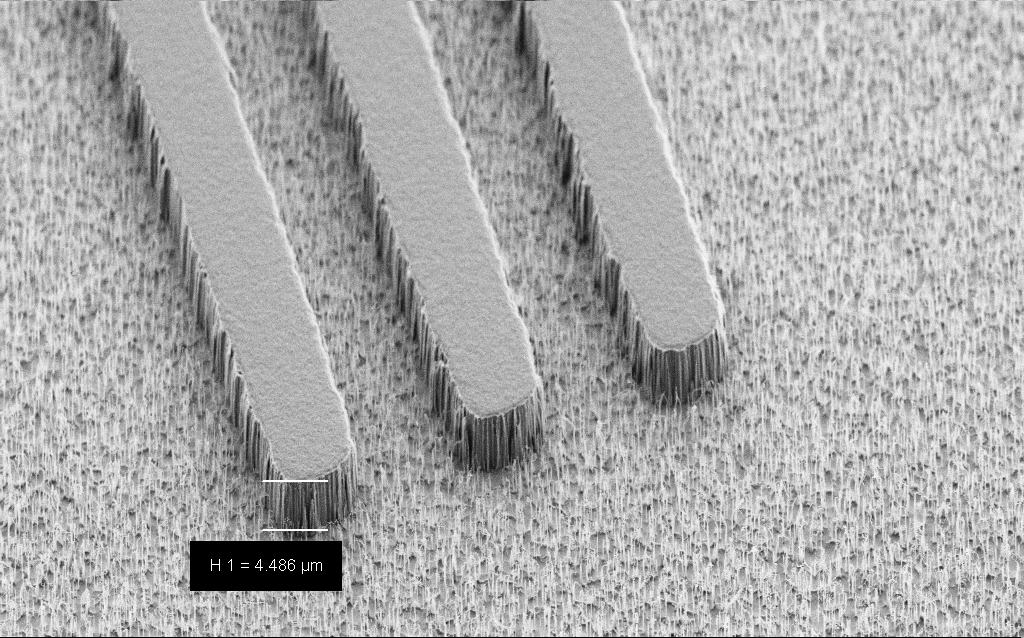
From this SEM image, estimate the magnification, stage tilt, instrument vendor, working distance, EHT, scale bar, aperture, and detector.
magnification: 4.01 K X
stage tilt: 45°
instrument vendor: Zeiss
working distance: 7 mm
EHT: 3 kV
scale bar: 10000 nm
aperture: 30 µm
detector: SE2